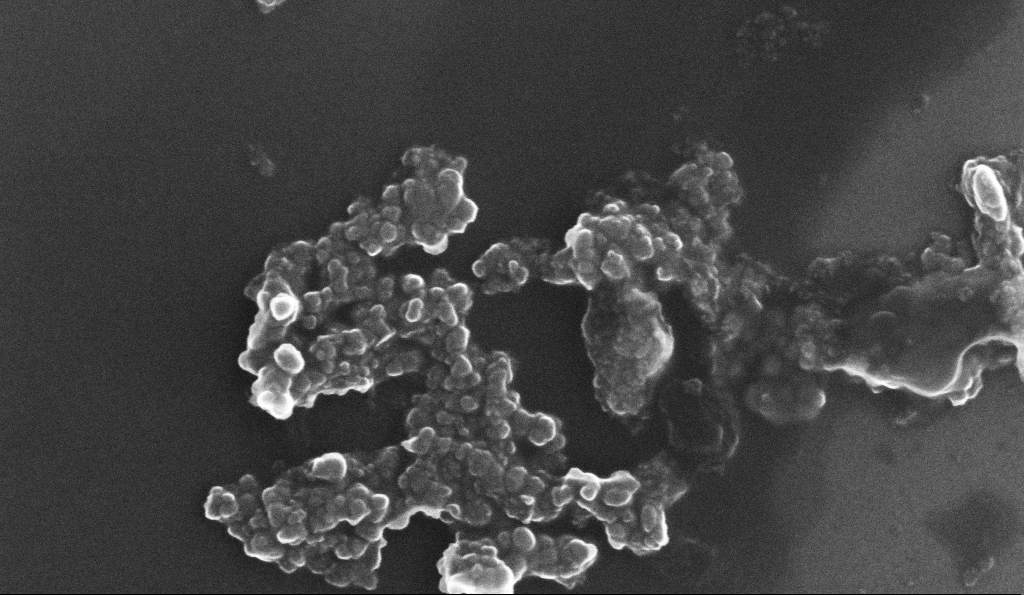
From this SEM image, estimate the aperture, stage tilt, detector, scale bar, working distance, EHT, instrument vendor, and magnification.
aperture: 30 µm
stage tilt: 0°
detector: InLens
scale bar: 200 nm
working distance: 5.3 mm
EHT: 10 kV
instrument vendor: Zeiss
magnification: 172.24 K X